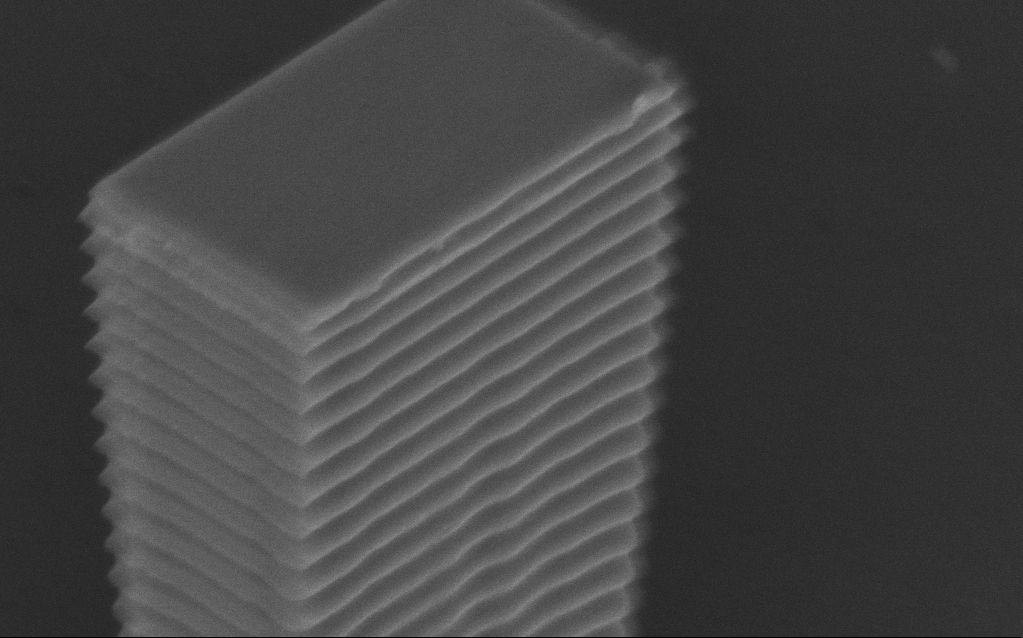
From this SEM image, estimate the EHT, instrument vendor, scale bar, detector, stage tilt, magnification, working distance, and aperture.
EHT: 5 kV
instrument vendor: Zeiss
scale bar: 1000 nm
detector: InLens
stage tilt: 50°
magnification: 42.11 K X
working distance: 9 mm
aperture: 30 µm